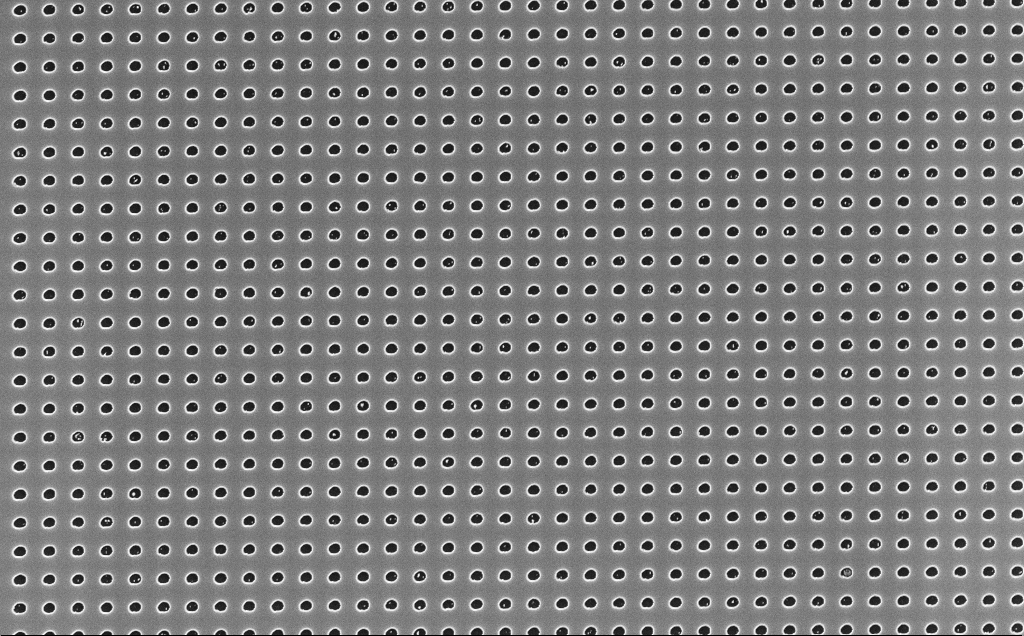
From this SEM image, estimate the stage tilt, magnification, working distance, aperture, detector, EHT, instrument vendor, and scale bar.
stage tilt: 0°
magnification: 10 K X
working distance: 4 mm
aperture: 30 µm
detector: InLens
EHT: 10 kV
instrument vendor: Zeiss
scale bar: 2000 nm